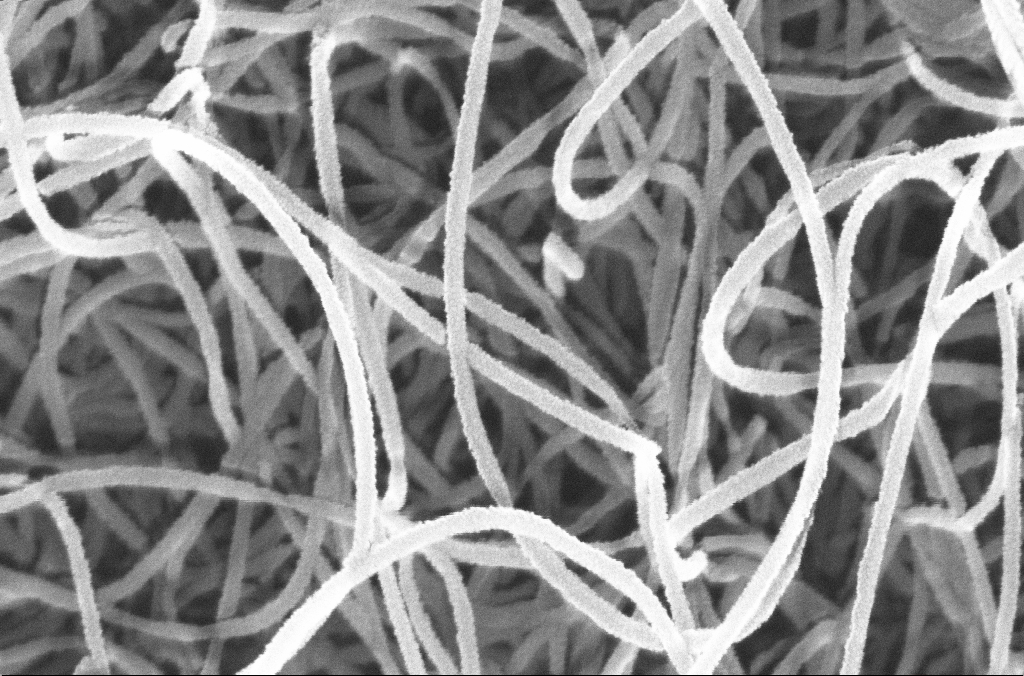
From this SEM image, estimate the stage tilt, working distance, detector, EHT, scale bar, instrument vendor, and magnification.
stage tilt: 0°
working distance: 4.5 mm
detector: InLens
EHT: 20 kV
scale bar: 100 nm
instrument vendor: Zeiss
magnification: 200 K X